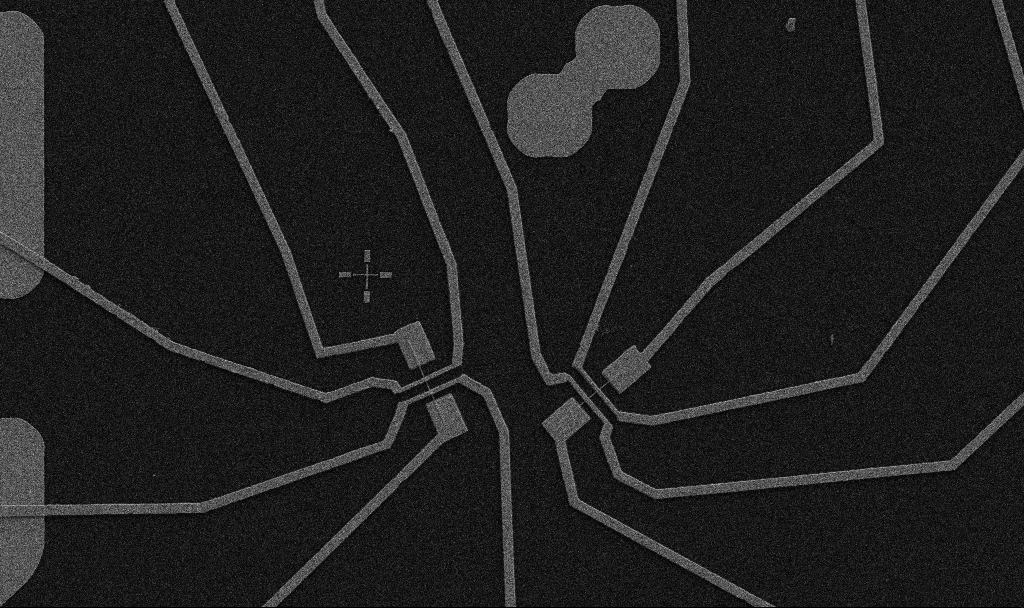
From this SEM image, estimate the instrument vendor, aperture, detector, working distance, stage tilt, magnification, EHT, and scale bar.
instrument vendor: Zeiss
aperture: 30 µm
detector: SE2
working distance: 10.7 mm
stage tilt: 0°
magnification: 5 K X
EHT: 5 kV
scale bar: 10000 nm